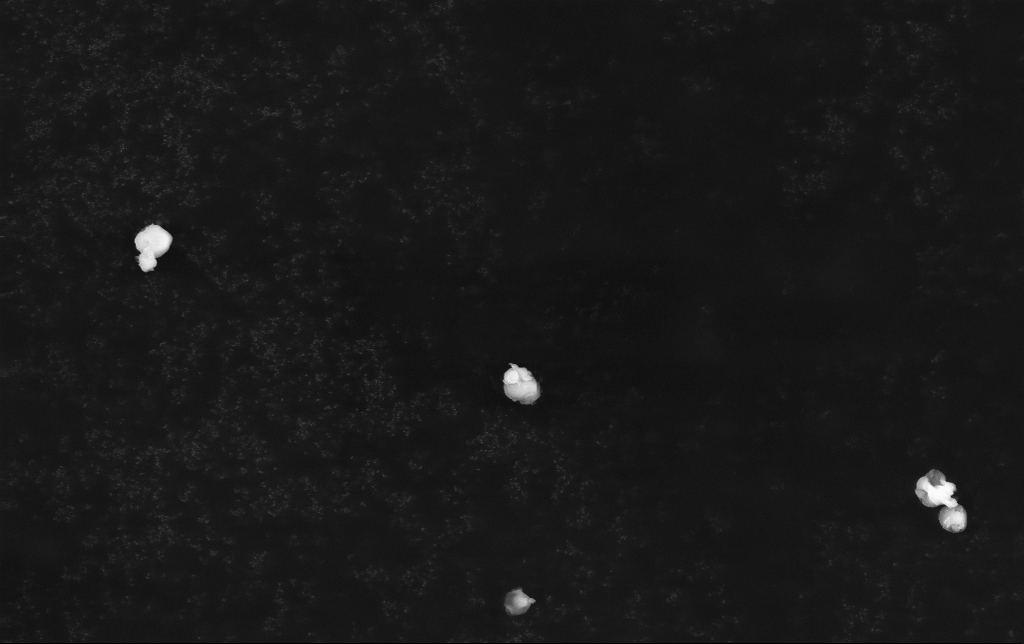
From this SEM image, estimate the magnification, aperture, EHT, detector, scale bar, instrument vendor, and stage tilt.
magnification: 3.93 K X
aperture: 30 µm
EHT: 15 kV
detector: InLens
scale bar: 10000 nm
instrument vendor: Zeiss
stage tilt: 0°